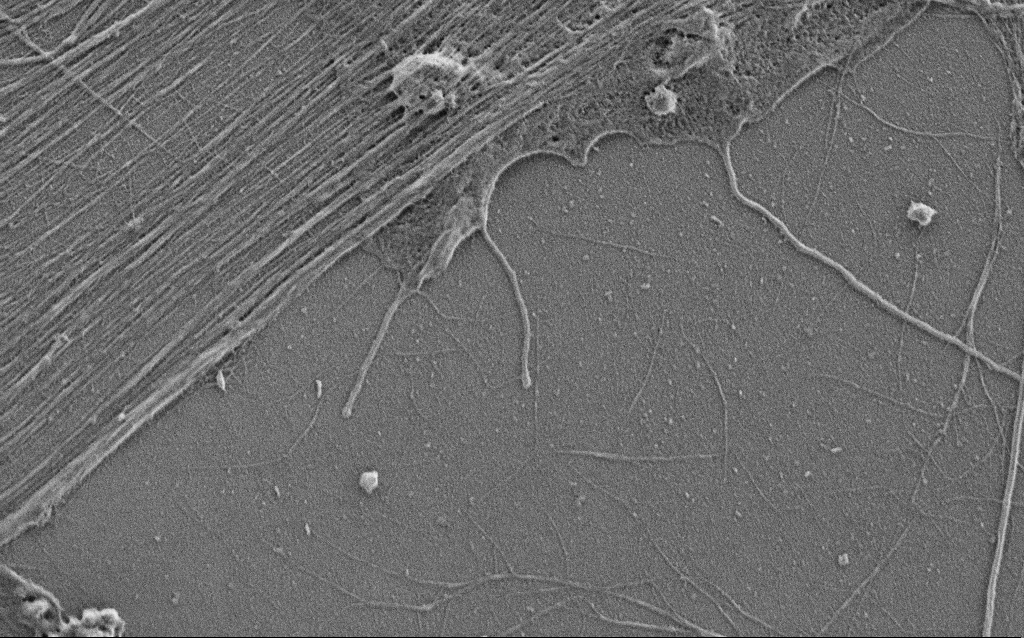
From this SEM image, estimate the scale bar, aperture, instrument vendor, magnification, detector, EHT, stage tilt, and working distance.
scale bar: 2000 nm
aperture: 30 µm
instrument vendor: Zeiss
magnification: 20 K X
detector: SE2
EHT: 0.9 kV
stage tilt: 0°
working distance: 4 mm